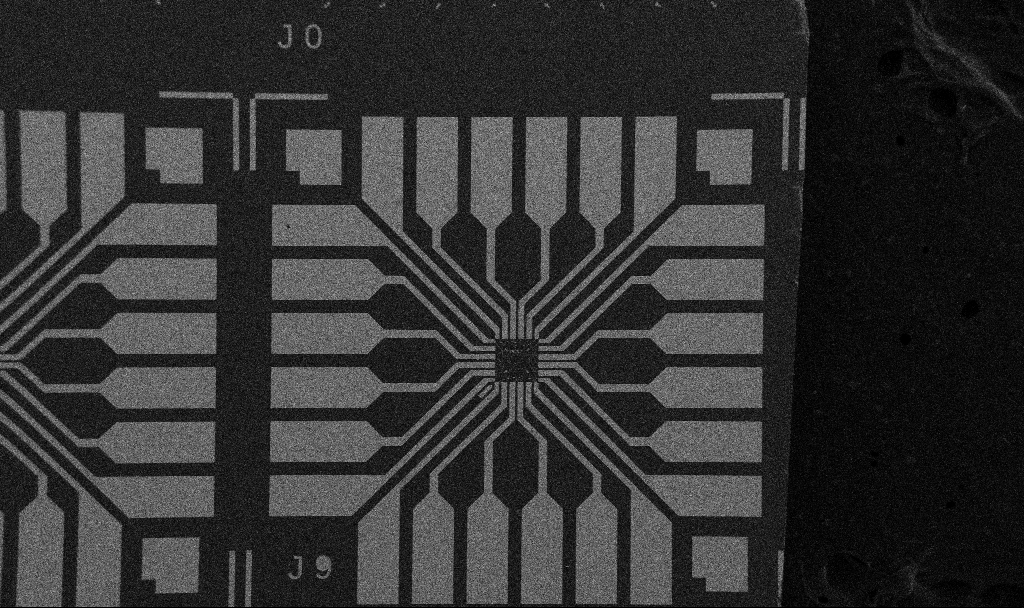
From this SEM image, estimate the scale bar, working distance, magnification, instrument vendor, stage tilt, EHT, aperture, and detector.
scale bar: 200000 nm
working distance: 10.7 mm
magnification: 0.1 K X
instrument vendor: Zeiss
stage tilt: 0°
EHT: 5 kV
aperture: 30 µm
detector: SE2